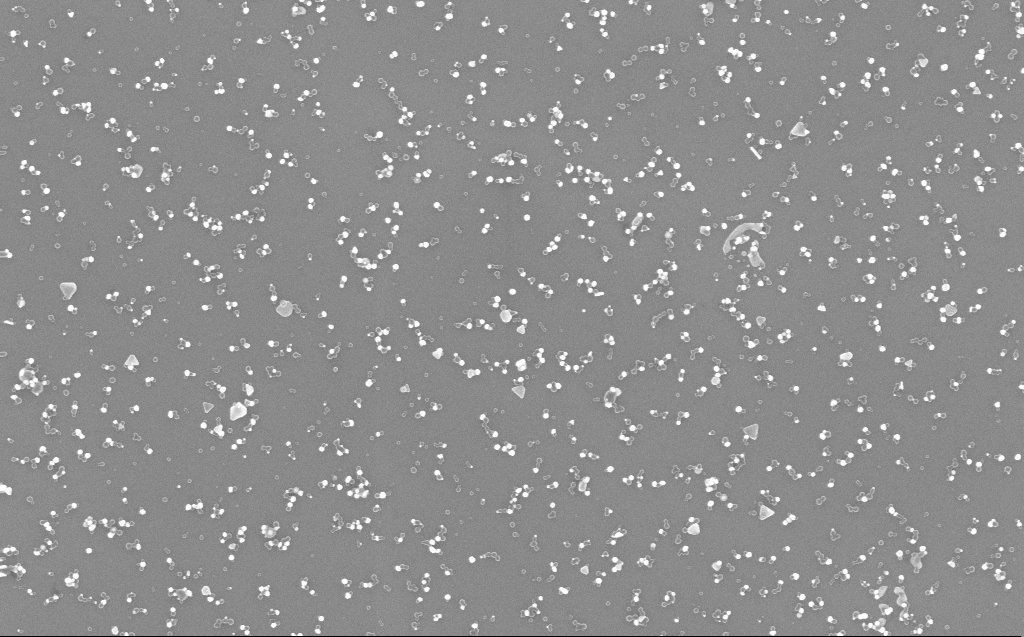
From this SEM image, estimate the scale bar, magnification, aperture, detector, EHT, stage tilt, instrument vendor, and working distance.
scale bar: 1000 nm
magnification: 40 K X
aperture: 30 µm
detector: InLens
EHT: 10 kV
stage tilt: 0°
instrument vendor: Zeiss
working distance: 3 mm